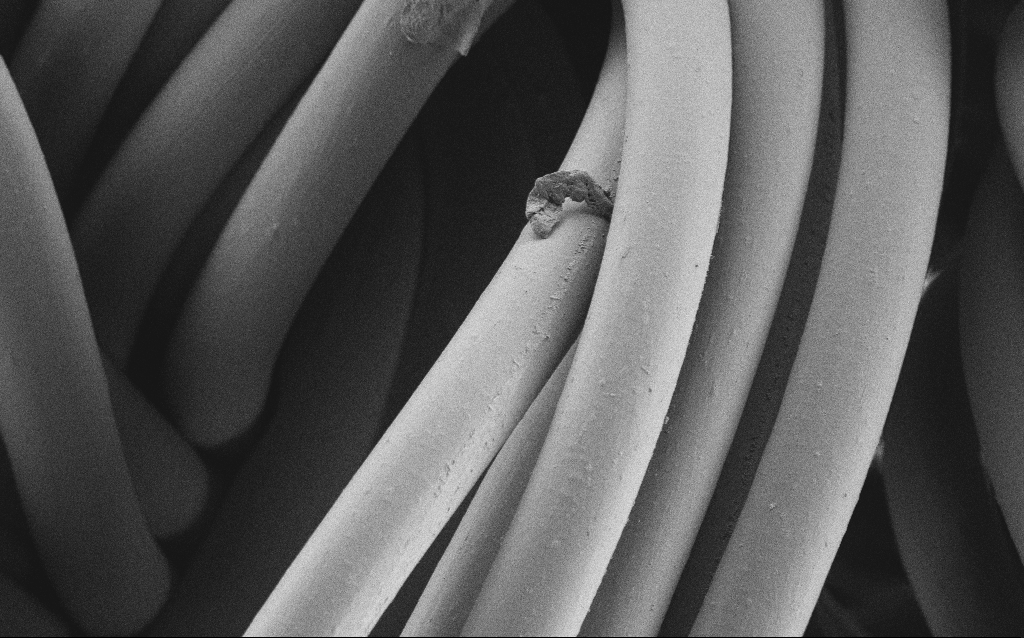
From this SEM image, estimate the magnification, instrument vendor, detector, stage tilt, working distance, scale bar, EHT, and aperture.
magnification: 1.61 K X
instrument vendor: Zeiss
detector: SE2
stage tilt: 0°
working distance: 4 mm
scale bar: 10000 nm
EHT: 1 kV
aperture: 30 µm